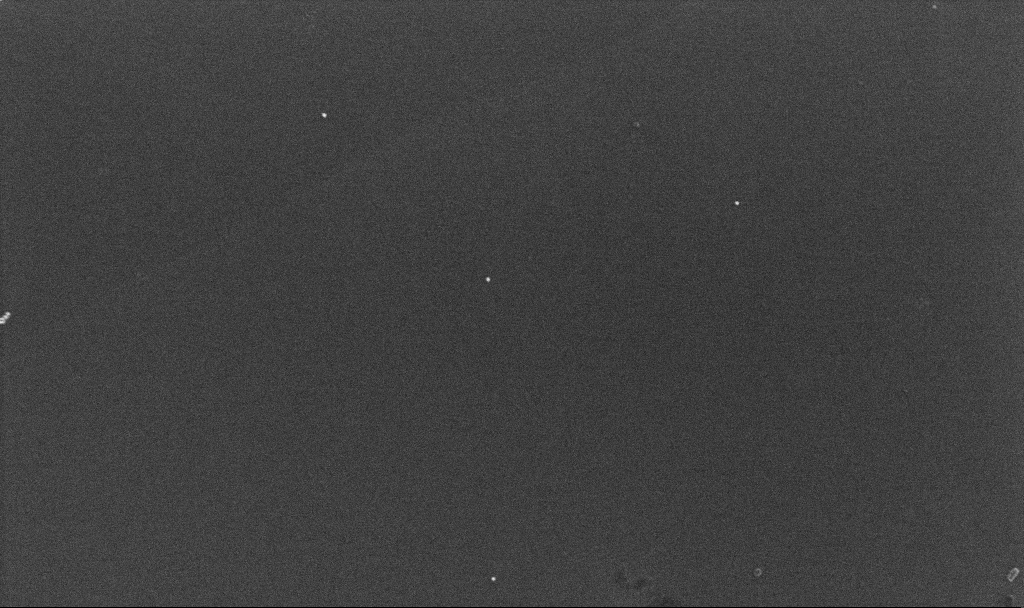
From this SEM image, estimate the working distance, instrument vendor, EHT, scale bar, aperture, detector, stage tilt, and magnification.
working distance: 4.3 mm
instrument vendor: Zeiss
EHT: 10 kV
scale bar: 1000 nm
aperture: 30 µm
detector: InLens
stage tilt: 0°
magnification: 66.83 K X